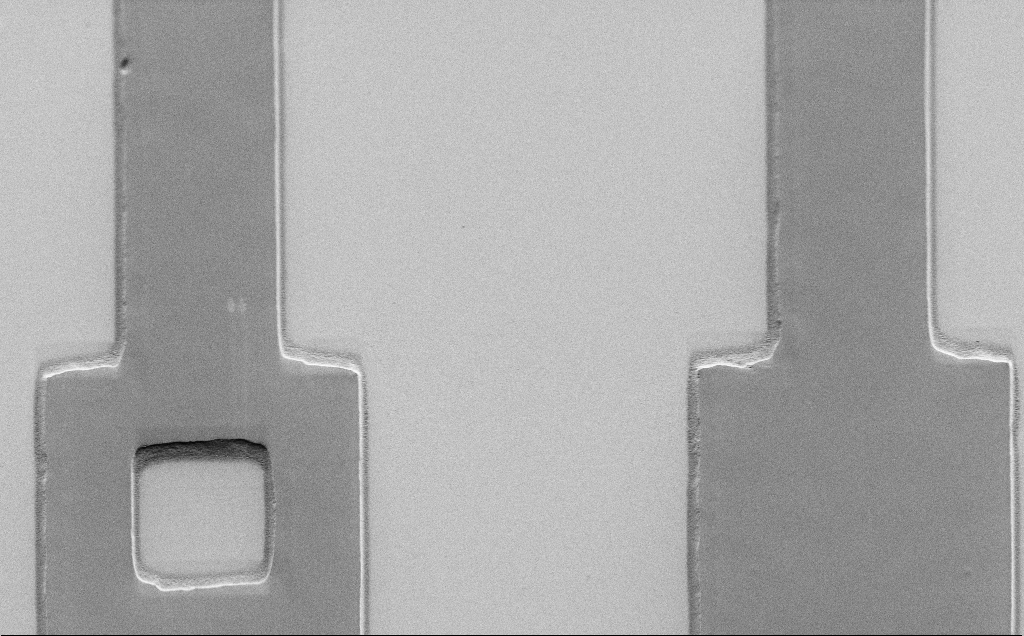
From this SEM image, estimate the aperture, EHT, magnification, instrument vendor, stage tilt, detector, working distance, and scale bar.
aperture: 30 µm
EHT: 1.5 kV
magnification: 2.39 K X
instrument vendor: Zeiss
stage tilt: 45°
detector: SE2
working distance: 7 mm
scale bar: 10000 nm